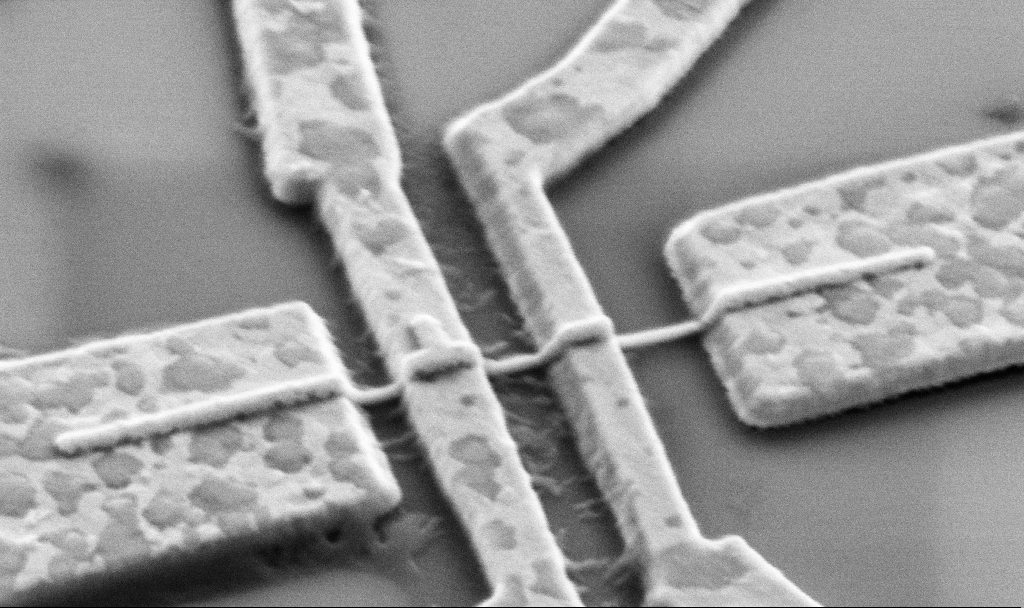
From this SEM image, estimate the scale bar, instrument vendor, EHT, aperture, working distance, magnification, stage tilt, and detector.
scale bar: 1000 nm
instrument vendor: Zeiss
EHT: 5 kV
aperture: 30 µm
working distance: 14.7 mm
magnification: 50 K X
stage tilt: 45°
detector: SE2